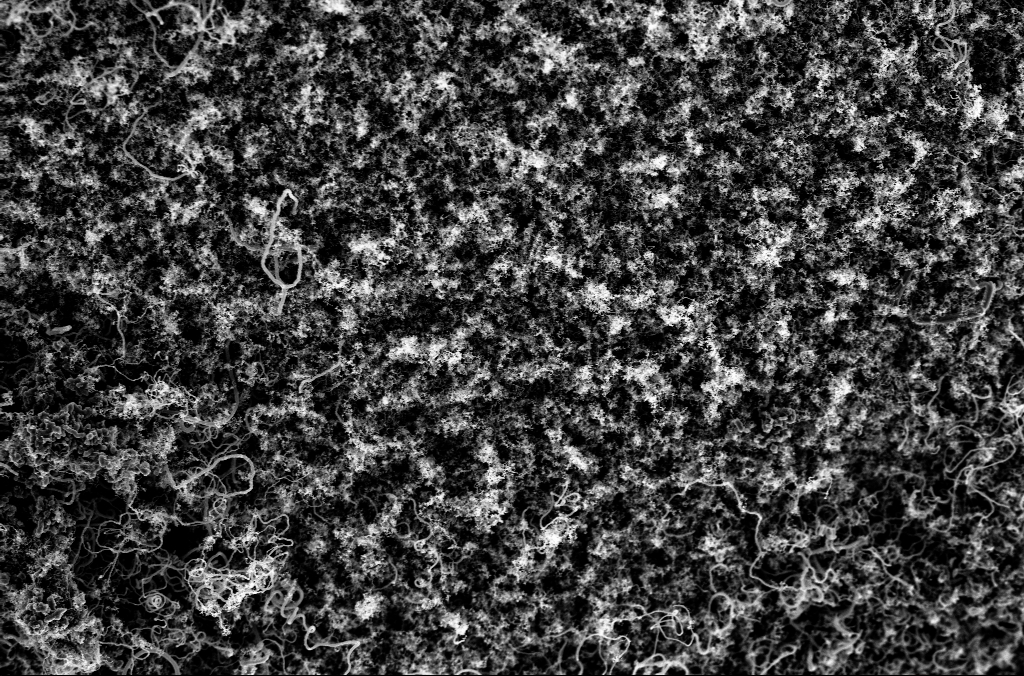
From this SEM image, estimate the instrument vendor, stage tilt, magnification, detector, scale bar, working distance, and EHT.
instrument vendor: Zeiss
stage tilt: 0°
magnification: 10 K X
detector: InLens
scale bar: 2000 nm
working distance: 5.1 mm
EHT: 3 kV